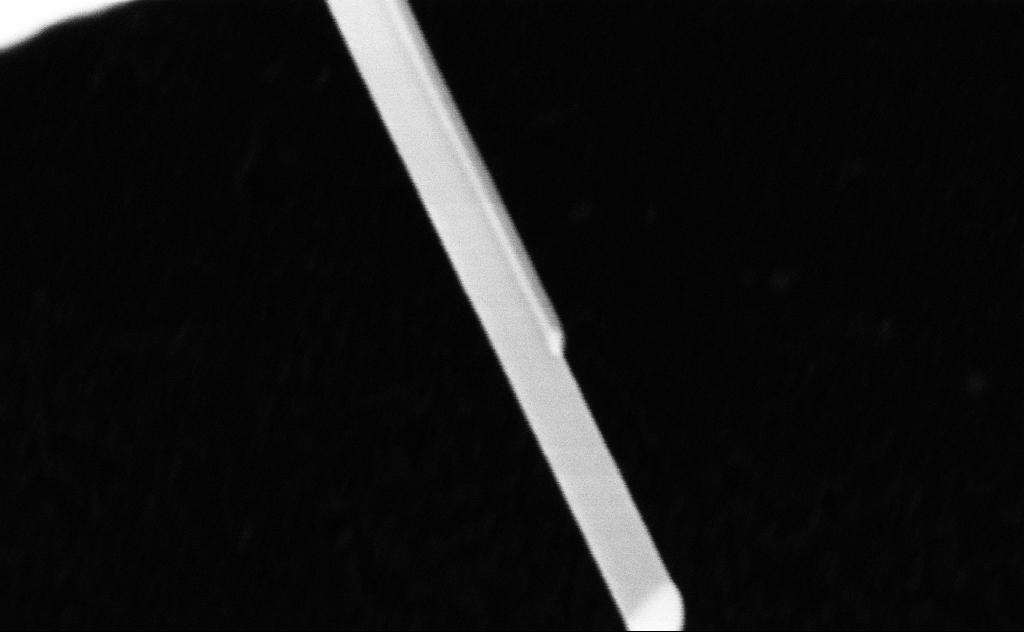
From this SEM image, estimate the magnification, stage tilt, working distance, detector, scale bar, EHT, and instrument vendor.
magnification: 330.16 K X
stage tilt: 0°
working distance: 8 mm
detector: InLens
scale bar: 200 nm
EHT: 20 kV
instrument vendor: Zeiss